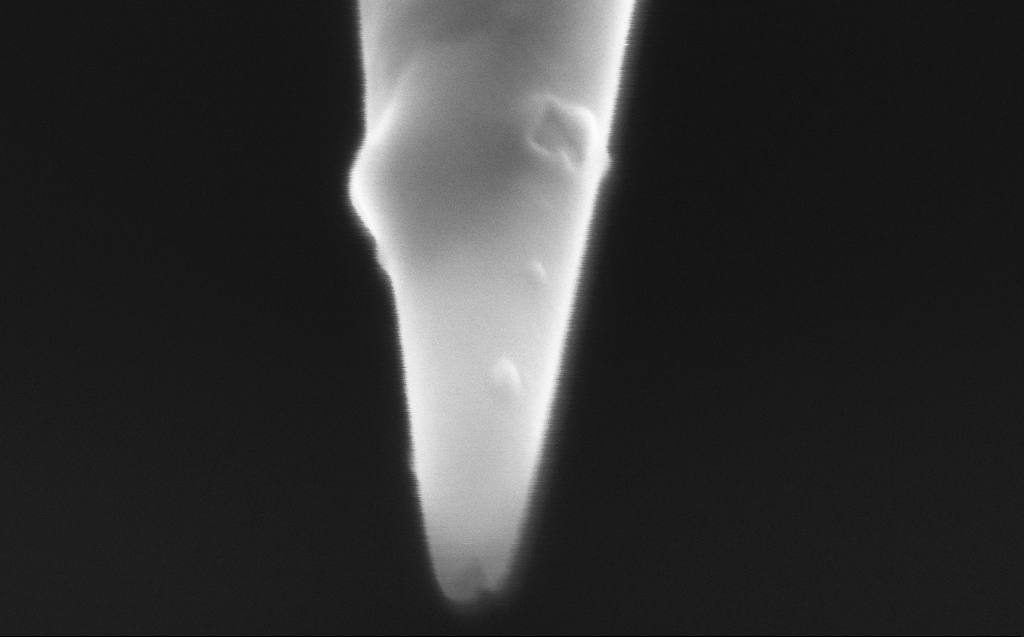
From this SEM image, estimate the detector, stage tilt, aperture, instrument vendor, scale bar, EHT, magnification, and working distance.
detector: InLens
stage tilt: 45.1°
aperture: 20 µm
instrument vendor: Zeiss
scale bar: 200 nm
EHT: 2.5 kV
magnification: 301.52 K X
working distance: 2 mm